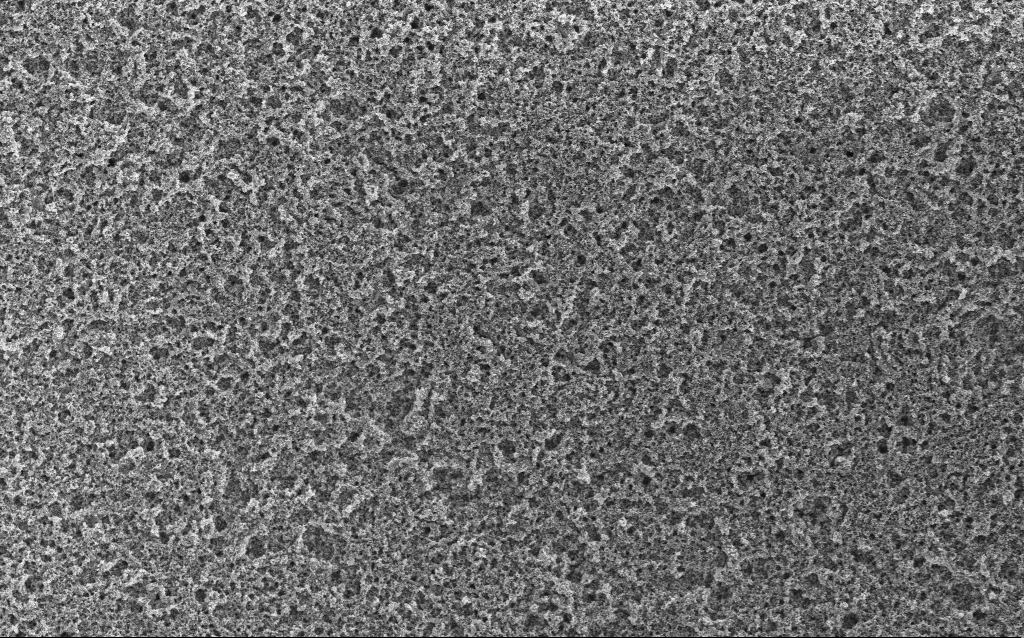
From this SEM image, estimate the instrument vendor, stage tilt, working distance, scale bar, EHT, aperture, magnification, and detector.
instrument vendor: Zeiss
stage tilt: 0°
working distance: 4.4 mm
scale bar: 10000 nm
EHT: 5 kV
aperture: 30 µm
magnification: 6.61 K X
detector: InLens